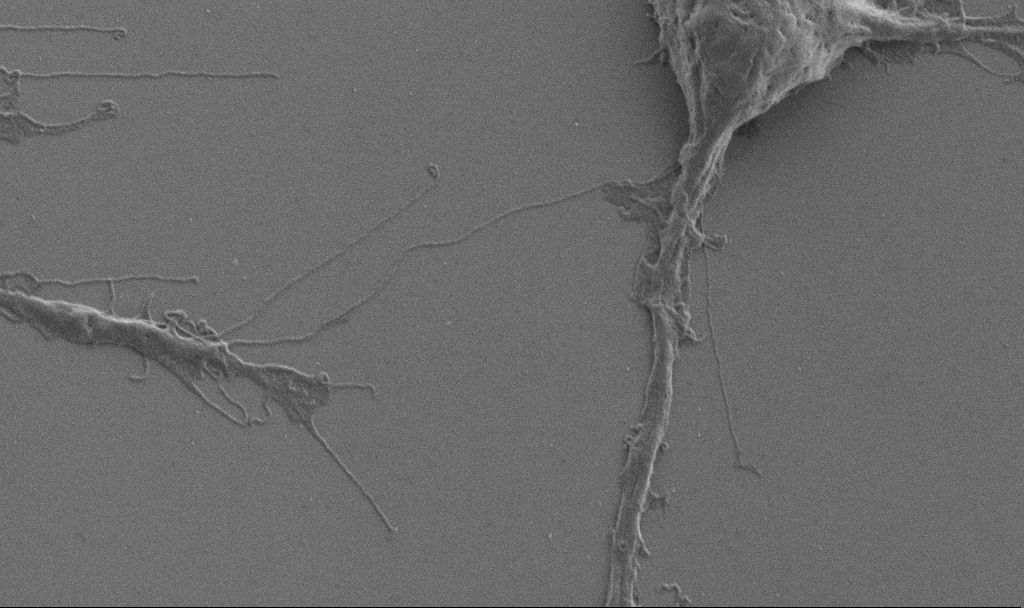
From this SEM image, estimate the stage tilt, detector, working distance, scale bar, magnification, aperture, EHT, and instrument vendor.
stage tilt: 0°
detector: SE2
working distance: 6.9 mm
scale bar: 2000 nm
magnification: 10 K X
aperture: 30 µm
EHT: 1 kV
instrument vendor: Zeiss